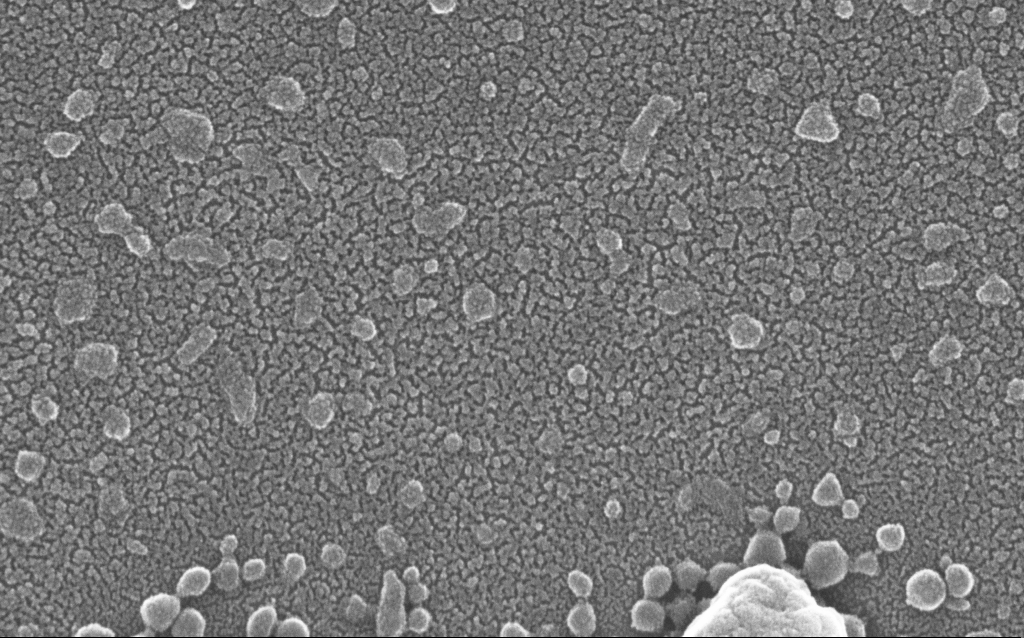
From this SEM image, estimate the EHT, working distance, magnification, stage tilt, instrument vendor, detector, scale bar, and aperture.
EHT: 20 kV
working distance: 1.5 mm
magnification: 300 K X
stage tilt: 0°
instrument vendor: Zeiss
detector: InLens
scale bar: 100 nm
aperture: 30 µm